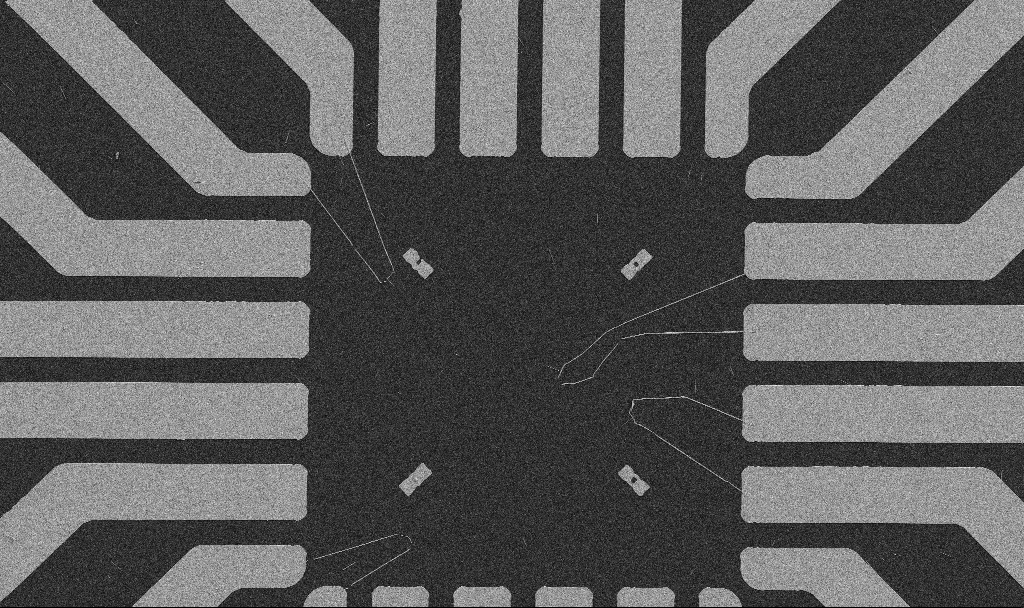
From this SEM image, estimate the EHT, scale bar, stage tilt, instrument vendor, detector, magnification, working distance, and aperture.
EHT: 5 kV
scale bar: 20000 nm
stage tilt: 0°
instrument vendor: Zeiss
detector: SE2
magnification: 1 K X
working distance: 10.7 mm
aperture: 30 µm